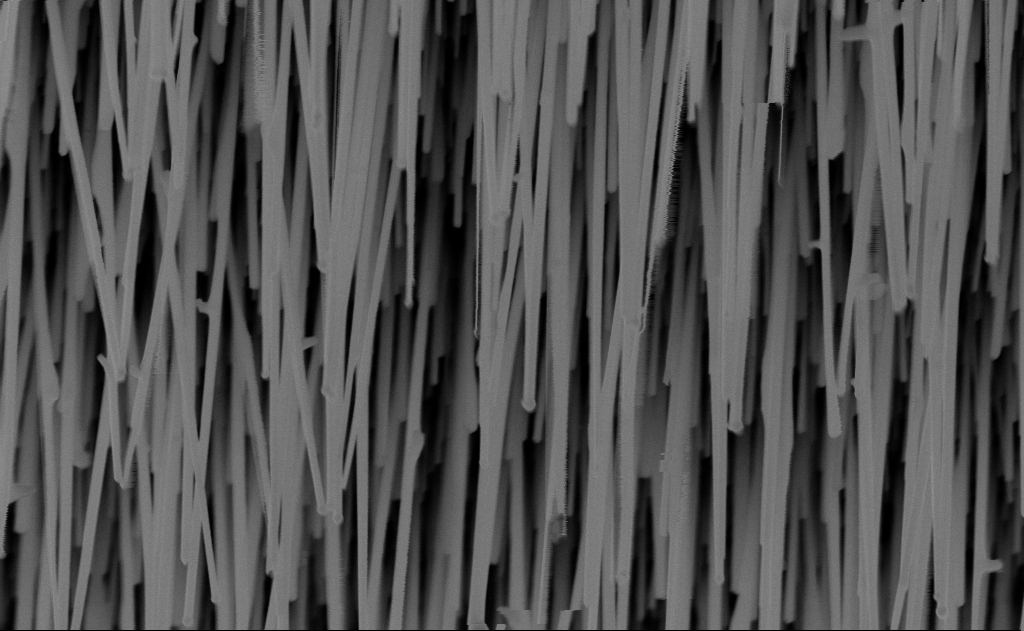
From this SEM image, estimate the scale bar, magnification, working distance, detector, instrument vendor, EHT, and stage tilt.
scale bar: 1000 nm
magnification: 40 K X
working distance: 9 mm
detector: InLens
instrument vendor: Zeiss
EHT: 10 kV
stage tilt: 0°